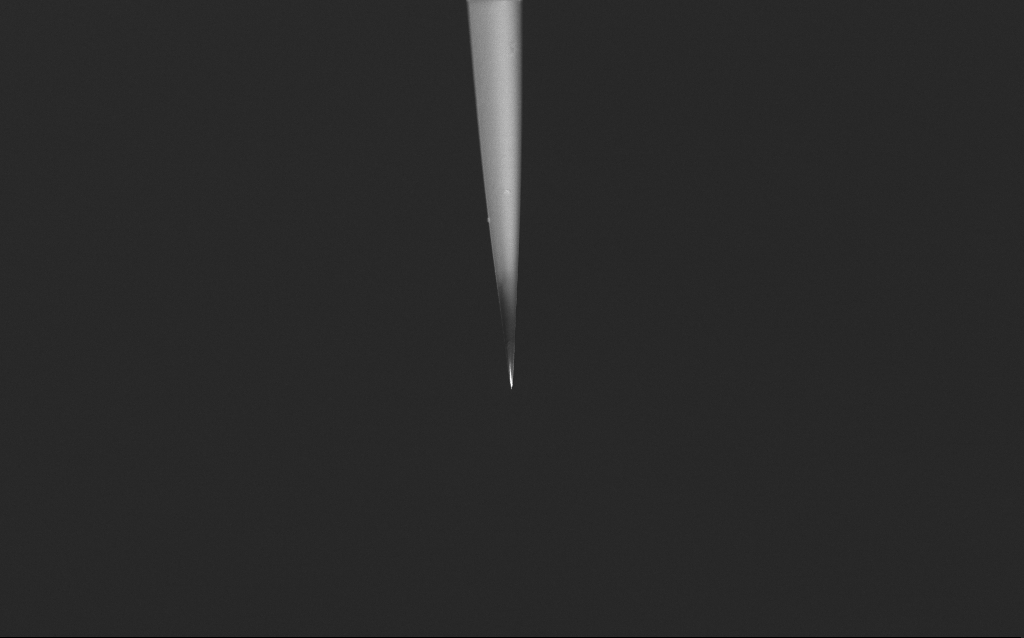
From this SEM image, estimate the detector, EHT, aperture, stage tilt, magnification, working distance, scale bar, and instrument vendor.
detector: InLens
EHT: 2.5 kV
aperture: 30 µm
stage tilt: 45°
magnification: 1 K X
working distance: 5 mm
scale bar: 20000 nm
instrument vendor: Zeiss